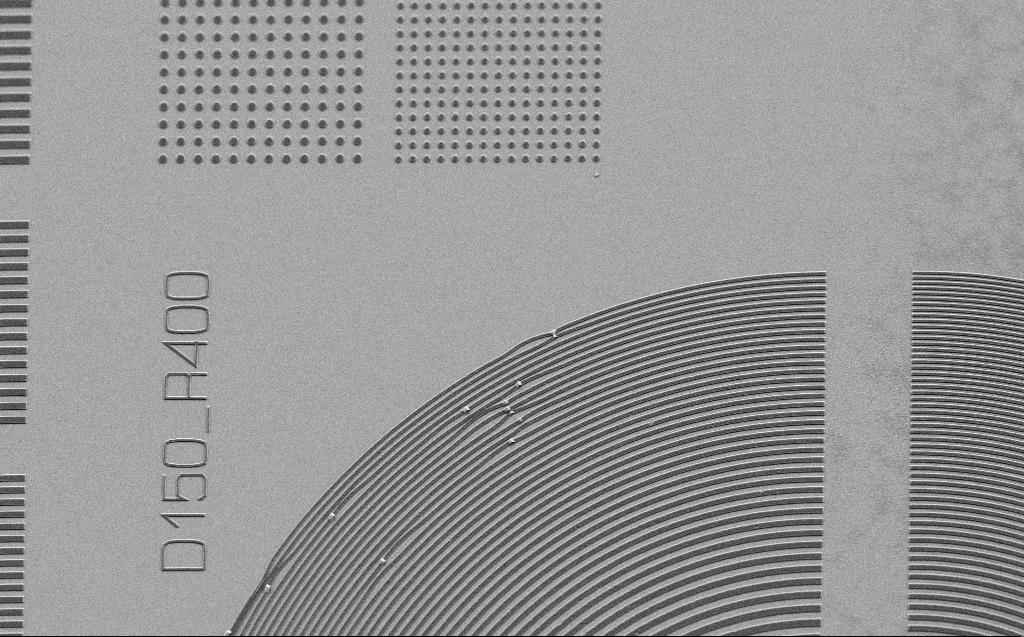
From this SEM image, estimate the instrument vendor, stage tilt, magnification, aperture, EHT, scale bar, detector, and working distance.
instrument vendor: Zeiss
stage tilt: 30°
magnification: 3.28 K X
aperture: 30 µm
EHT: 2.5 kV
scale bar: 10000 nm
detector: SE2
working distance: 5 mm